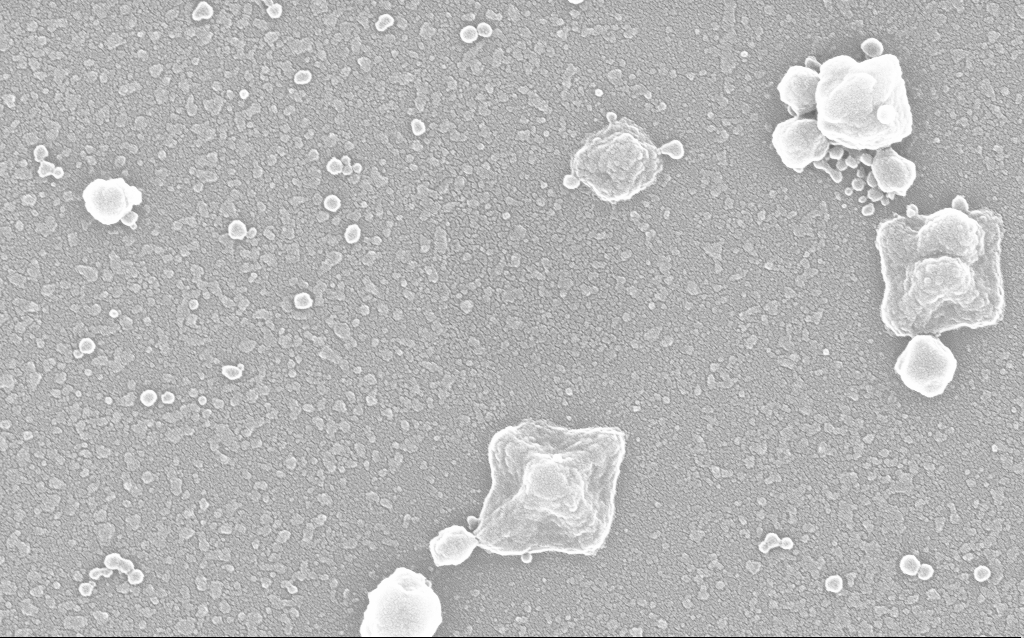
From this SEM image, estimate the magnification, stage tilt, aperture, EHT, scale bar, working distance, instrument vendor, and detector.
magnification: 100 K X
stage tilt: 0°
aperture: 30 µm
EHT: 20 kV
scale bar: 200 nm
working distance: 1.8 mm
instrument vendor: Zeiss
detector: InLens